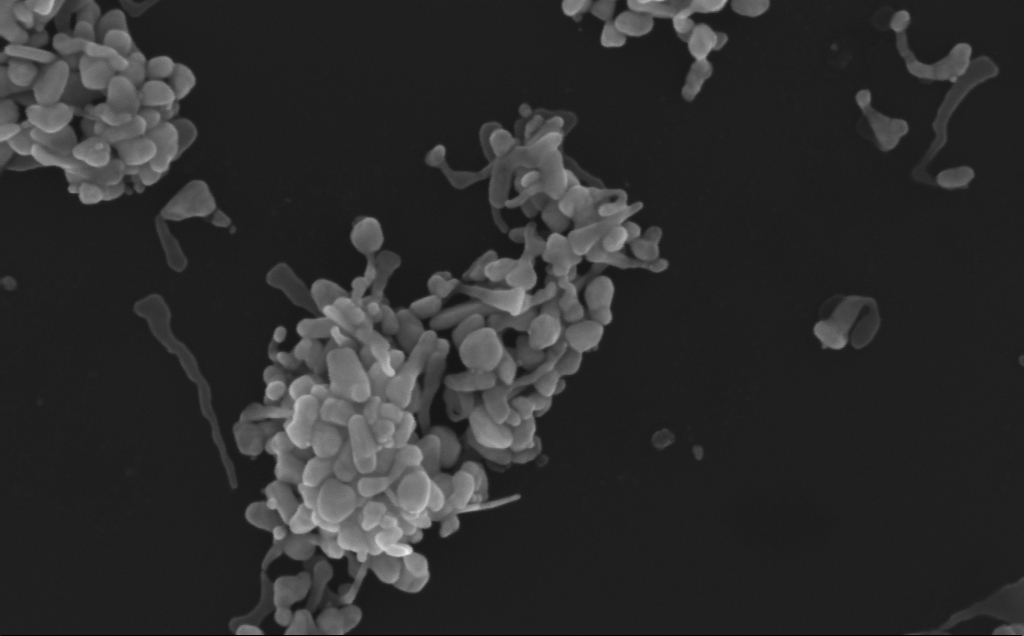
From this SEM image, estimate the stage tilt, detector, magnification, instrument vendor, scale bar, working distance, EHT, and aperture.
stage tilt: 0°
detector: InLens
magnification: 188.8 K X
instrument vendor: Zeiss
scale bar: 200 nm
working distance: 3 mm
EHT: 10 kV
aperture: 30 µm